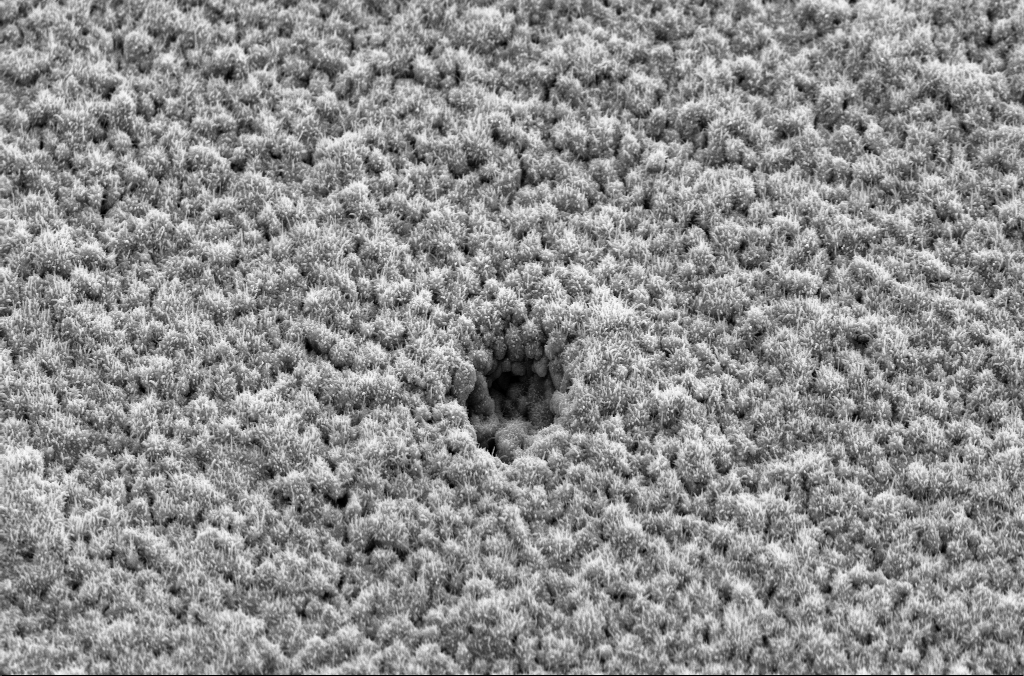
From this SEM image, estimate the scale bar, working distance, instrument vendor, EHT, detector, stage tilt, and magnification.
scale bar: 2000 nm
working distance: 8 mm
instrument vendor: Zeiss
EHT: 10 kV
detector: SE2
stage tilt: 45°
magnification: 9.13 K X